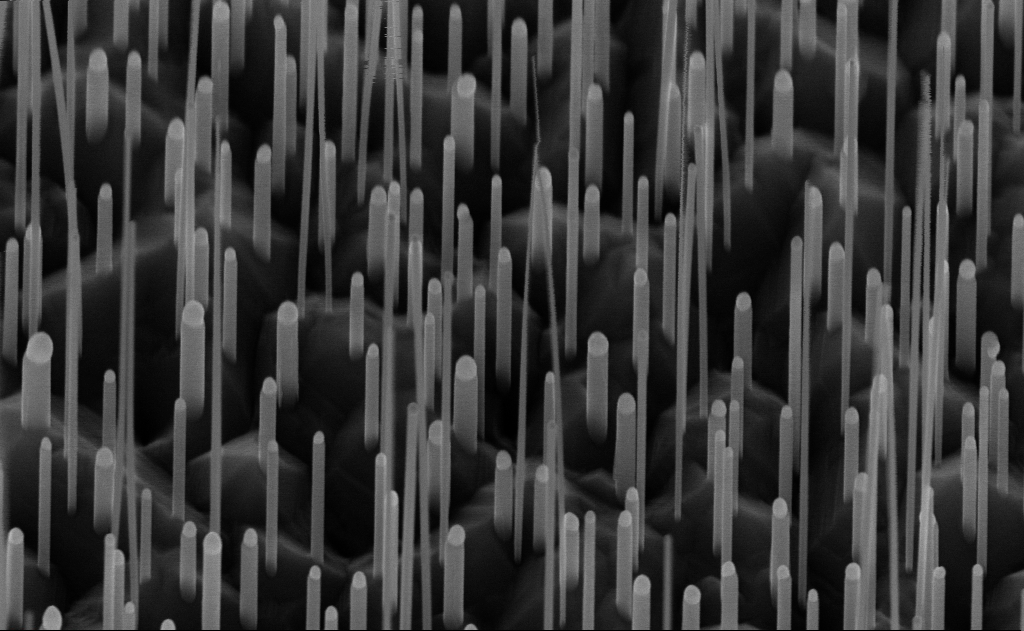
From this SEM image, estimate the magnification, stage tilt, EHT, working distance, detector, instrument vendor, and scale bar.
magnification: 80 K X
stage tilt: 45°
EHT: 10 kV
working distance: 6 mm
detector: InLens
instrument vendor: Zeiss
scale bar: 200 nm